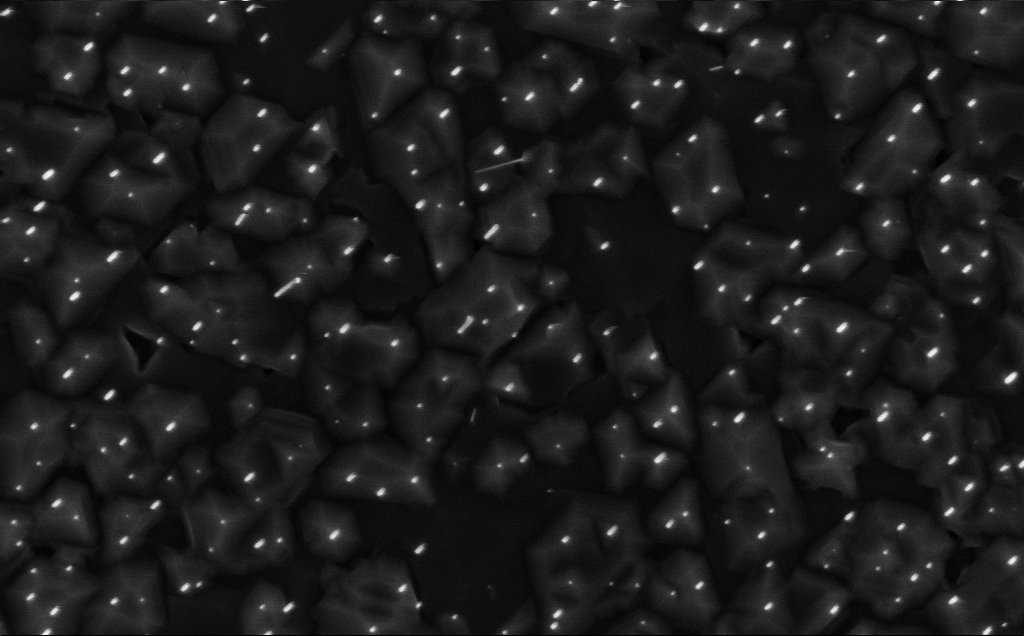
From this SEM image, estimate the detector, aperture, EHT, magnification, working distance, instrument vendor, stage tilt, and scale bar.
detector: InLens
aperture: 30 µm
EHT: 7 kV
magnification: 40 K X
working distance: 4 mm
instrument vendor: Zeiss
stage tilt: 0°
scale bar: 1000 nm